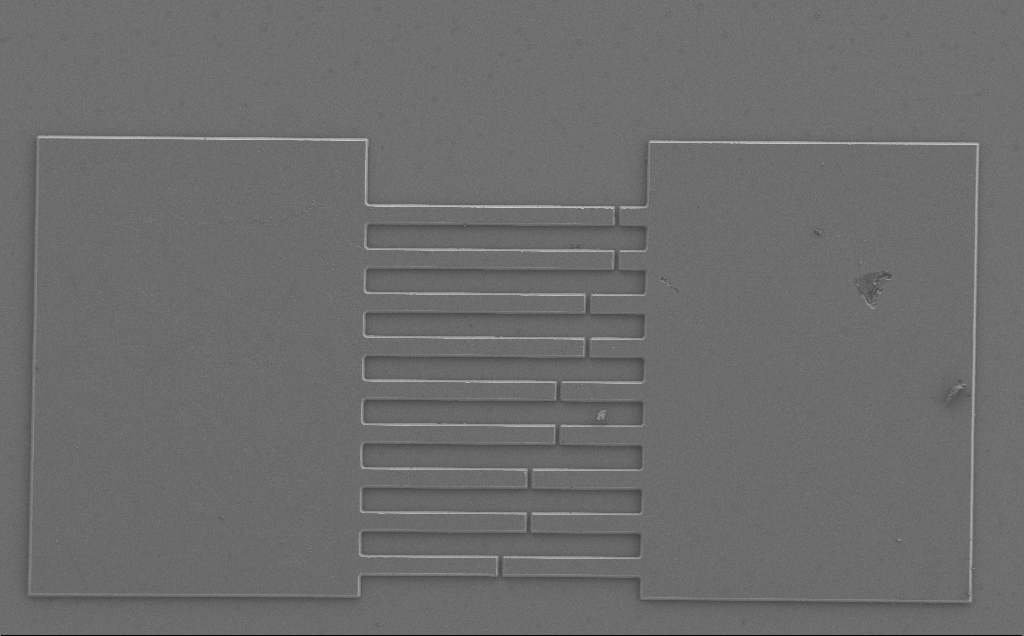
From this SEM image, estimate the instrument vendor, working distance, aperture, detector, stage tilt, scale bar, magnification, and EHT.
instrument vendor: Zeiss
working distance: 6 mm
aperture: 30 µm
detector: SE2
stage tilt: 0°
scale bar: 100000 nm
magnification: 0.409 K X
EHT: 3 kV